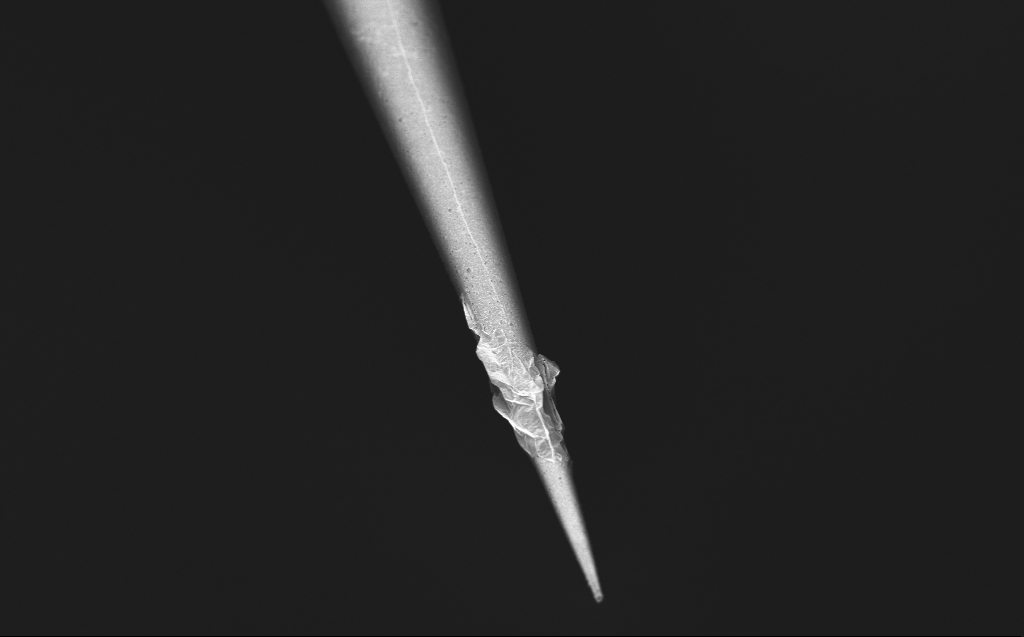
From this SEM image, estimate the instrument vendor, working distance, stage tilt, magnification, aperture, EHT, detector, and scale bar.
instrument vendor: Zeiss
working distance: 4 mm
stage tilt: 45°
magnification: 5 K X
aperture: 30 µm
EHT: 1 kV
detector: InLens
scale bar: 10000 nm